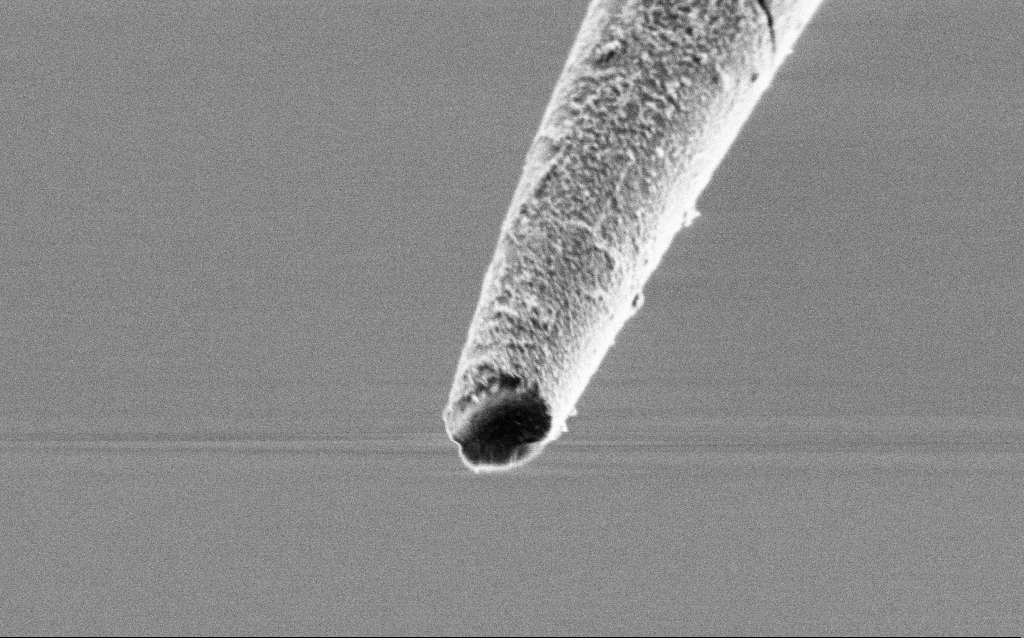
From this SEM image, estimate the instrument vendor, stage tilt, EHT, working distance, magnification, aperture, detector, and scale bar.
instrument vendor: Zeiss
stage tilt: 45°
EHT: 1 kV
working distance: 6.7 mm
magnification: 50 K X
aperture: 30 µm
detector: SE2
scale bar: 1000 nm